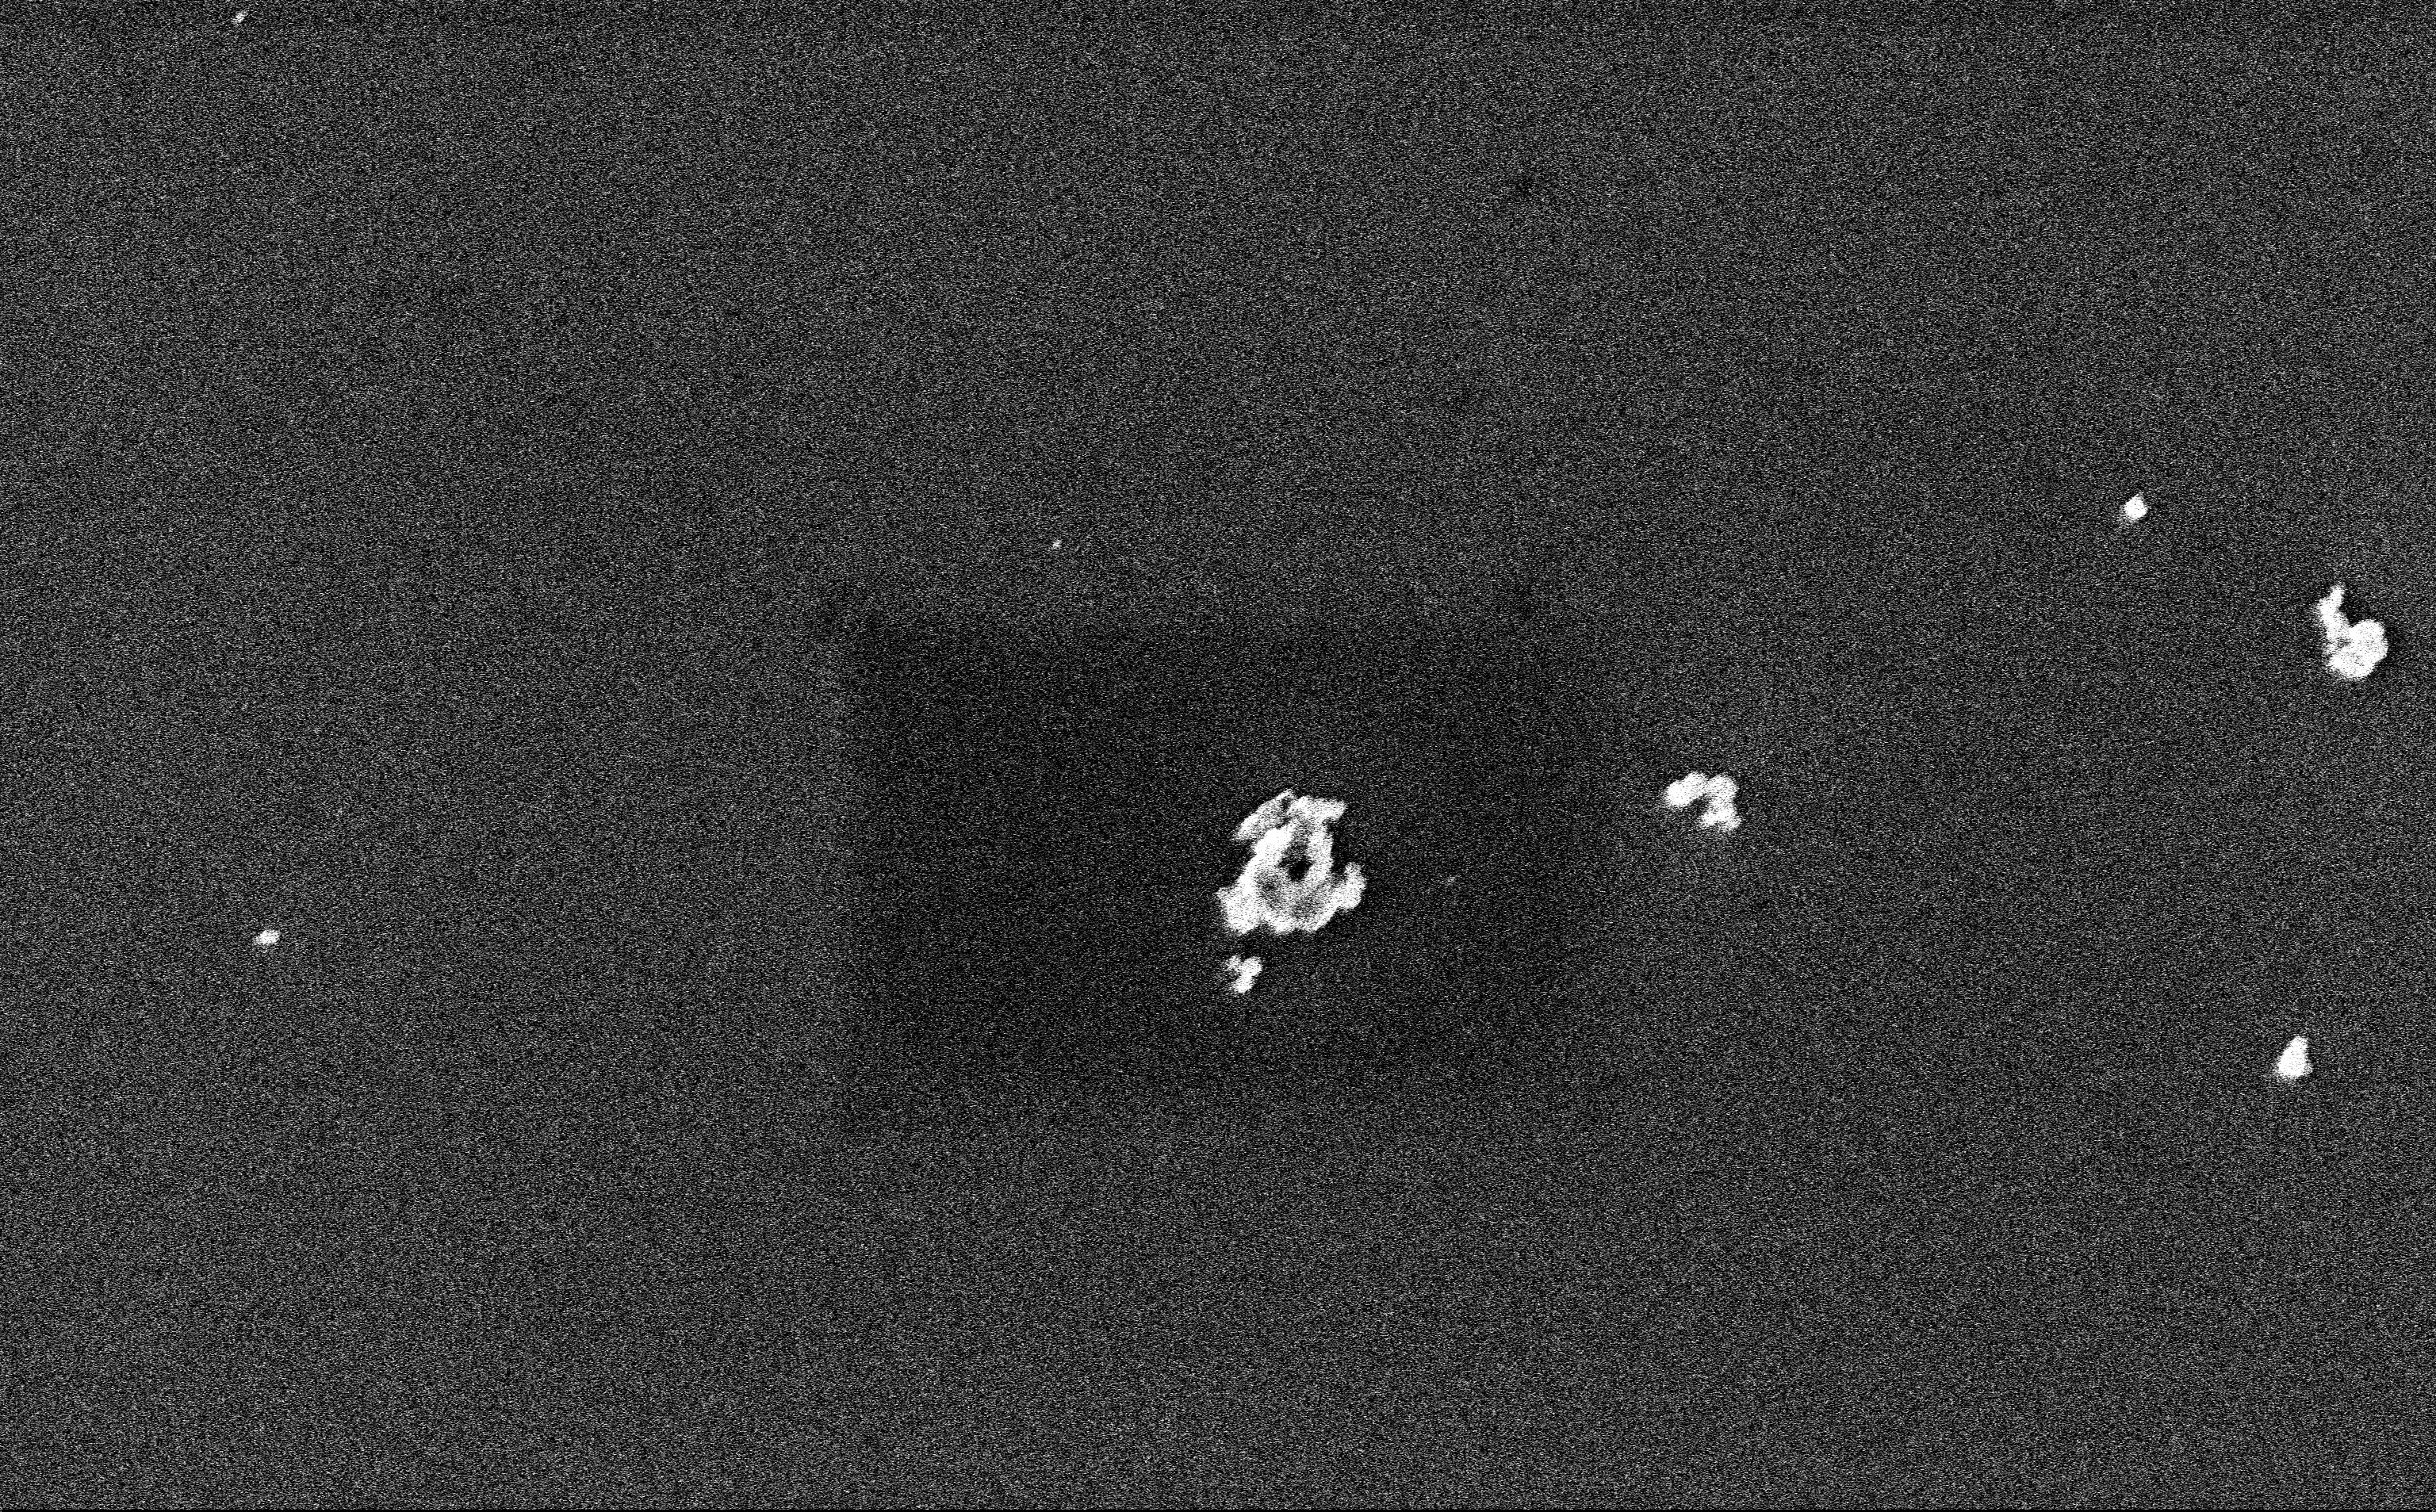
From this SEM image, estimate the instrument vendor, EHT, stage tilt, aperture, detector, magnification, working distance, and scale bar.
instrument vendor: Zeiss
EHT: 3 kV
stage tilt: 0°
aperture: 30 µm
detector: InLens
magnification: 27.35 K X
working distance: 3 mm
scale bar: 1000 nm